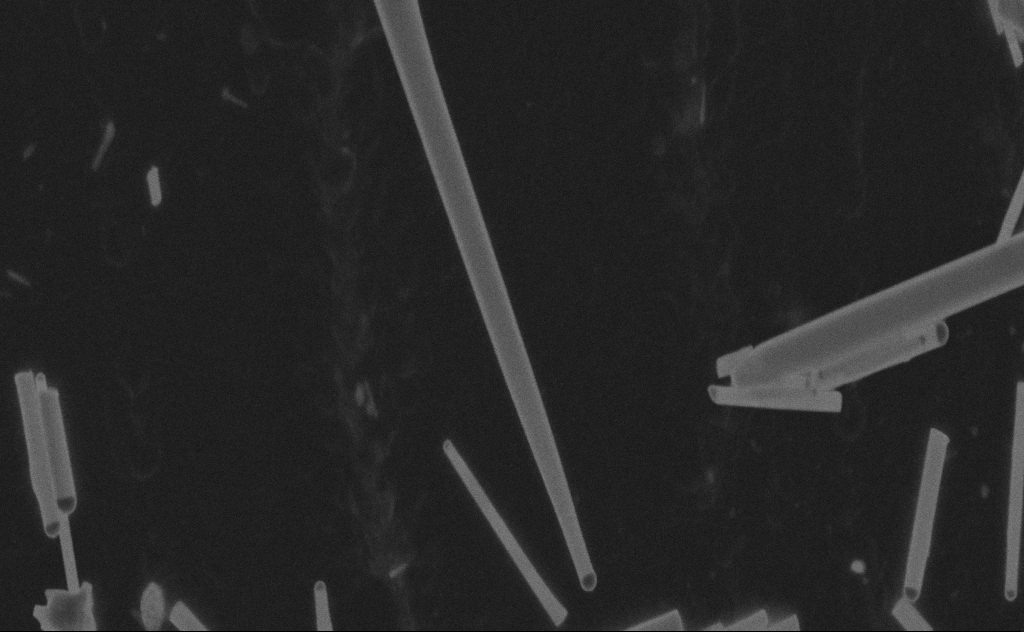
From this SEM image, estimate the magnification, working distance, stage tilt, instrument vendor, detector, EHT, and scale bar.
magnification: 102.58 K X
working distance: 9 mm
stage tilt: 0°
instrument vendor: Zeiss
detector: SE2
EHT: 20 kV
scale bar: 200 nm